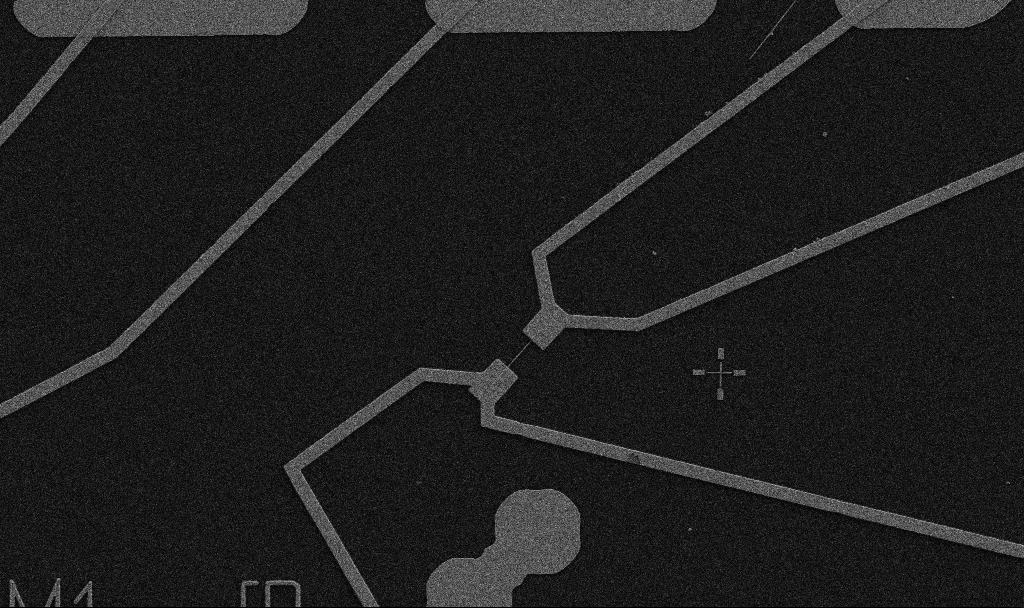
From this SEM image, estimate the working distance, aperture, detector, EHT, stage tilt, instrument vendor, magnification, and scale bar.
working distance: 10.7 mm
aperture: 30 µm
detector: SE2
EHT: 5 kV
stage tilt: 0°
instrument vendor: Zeiss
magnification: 5 K X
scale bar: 10000 nm